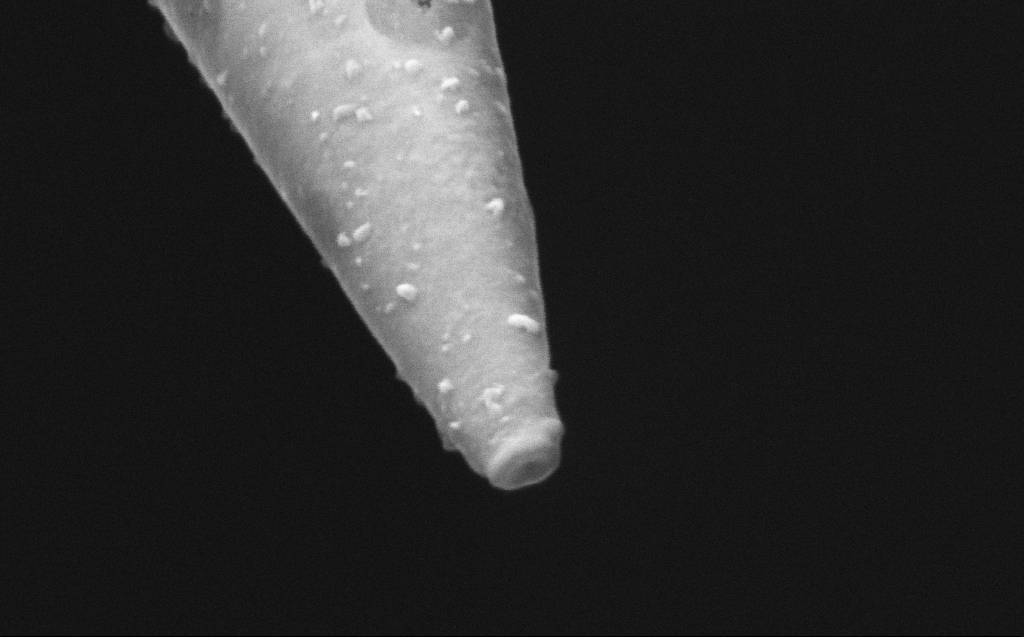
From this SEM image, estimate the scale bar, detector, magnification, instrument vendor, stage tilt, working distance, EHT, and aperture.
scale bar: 200 nm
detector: InLens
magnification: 250 K X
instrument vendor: Zeiss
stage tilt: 45°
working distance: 6 mm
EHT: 5 kV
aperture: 30 µm